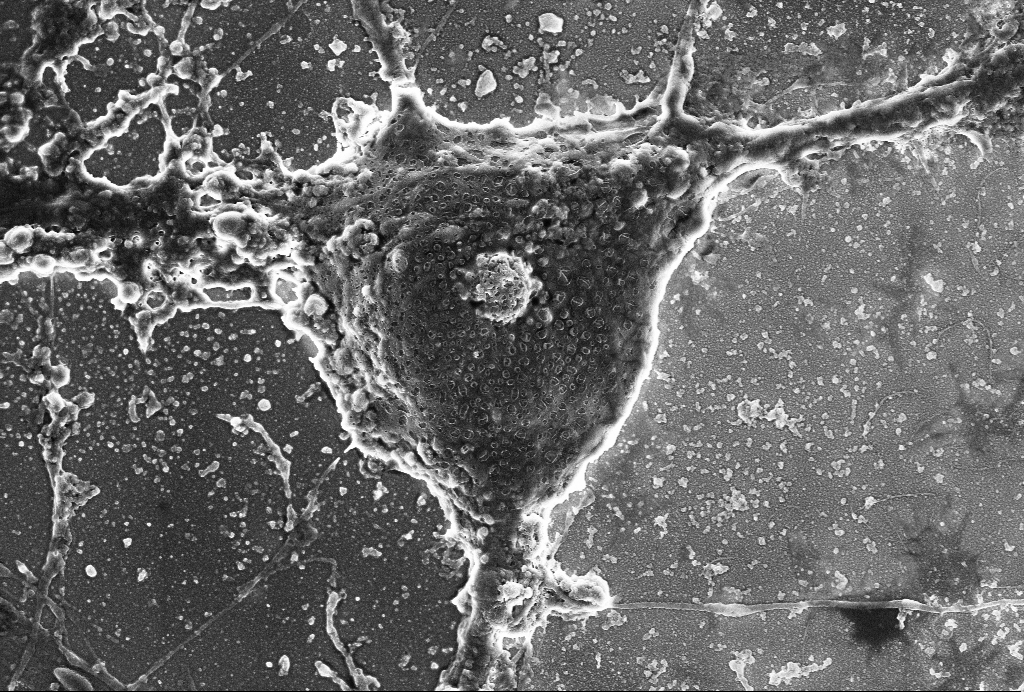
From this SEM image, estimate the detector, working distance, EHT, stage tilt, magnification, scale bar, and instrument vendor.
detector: SE2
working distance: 6 mm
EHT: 4 kV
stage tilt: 0°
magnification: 10 K X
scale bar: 2000 nm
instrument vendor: Zeiss